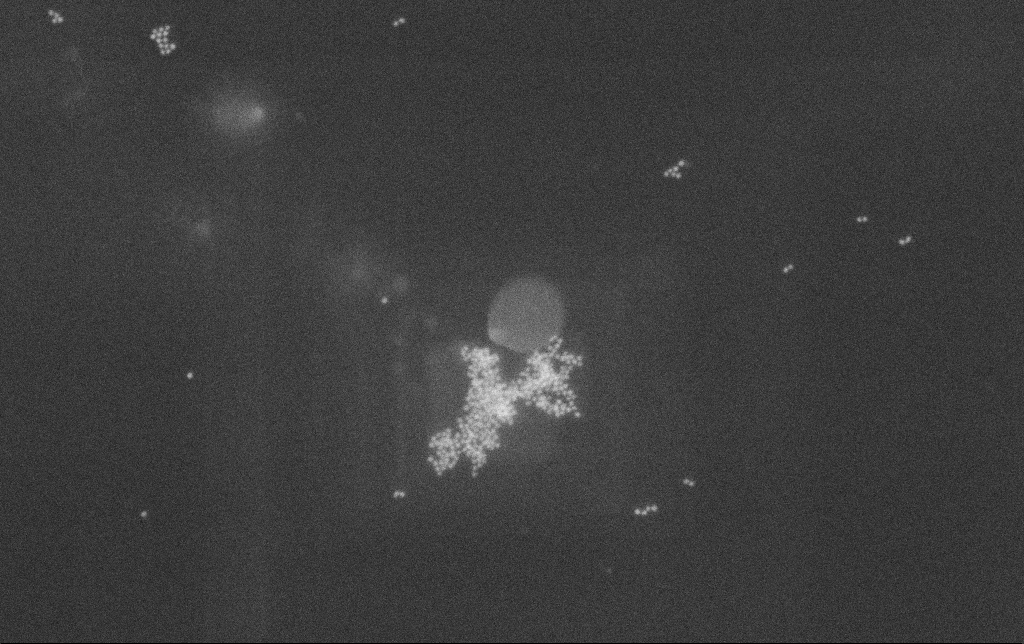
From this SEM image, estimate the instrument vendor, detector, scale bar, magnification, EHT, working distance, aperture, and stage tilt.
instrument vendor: Zeiss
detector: SE2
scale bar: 100 nm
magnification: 149.16 K X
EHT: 30 kV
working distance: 10.8 mm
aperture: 20 µm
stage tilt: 0°